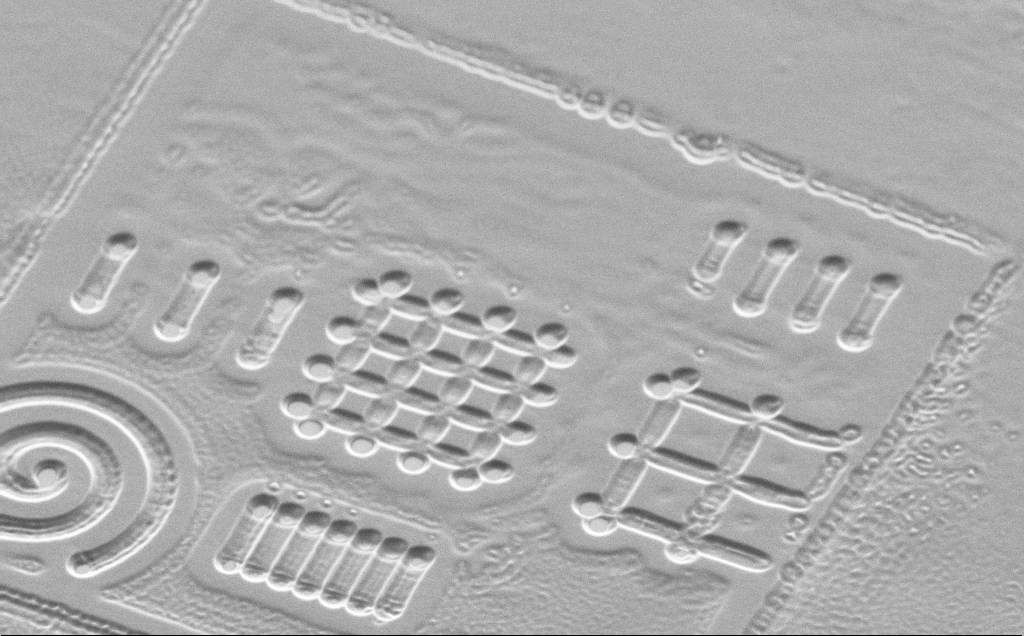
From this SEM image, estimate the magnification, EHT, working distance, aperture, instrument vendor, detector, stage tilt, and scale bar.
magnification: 3.29 K X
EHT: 5 kV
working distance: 10 mm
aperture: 30 µm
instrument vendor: Zeiss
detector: SE2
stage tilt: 45°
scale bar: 20000 nm